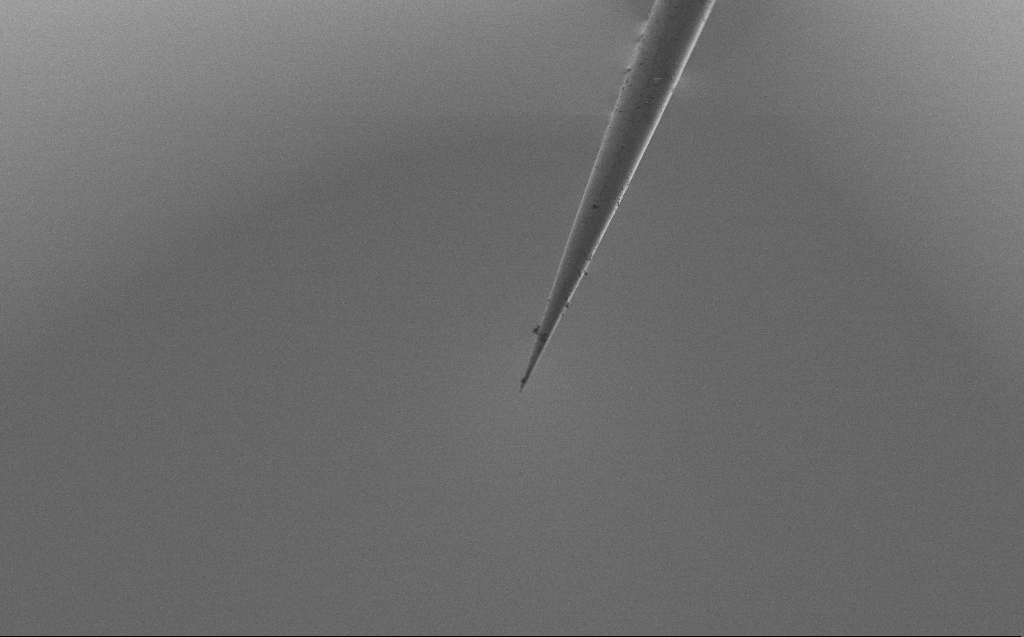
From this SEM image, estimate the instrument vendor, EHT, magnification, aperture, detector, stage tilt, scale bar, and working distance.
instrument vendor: Zeiss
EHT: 2 kV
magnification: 1 K X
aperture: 30 µm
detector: SE2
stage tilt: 45°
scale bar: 20000 nm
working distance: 3 mm